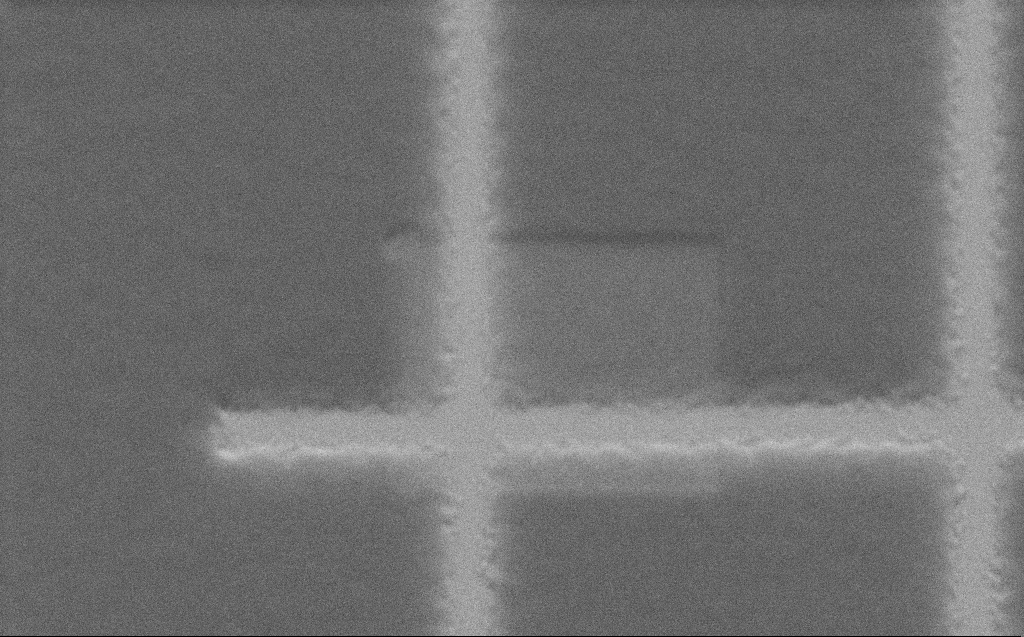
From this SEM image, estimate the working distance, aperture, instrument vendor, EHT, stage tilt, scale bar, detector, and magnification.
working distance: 5 mm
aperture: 30 µm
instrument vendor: Zeiss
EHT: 3 kV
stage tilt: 45°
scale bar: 2000 nm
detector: SE2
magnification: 18.78 K X